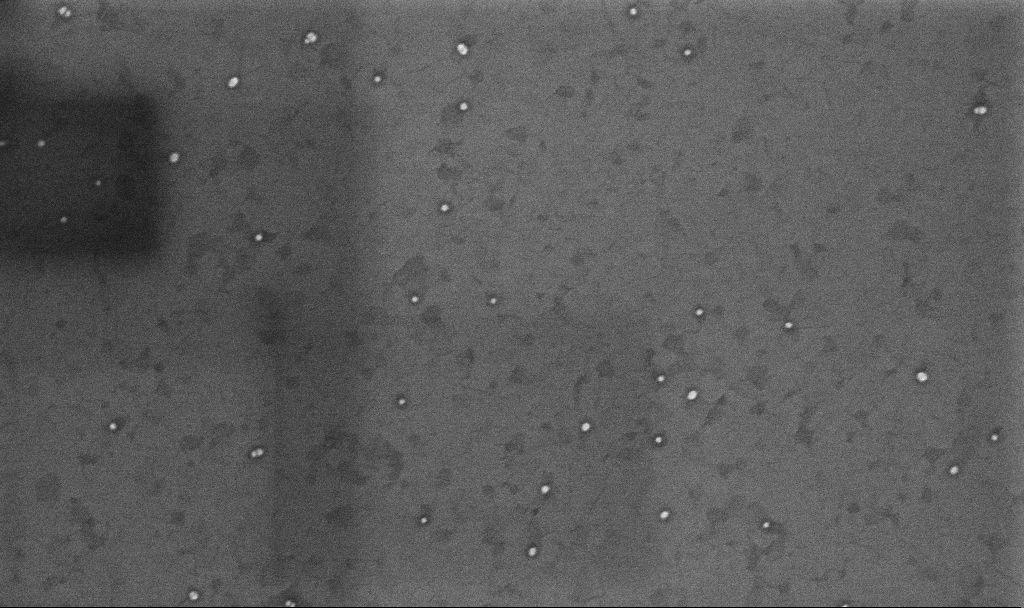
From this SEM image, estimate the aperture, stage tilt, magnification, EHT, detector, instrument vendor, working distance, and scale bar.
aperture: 30 µm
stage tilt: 0°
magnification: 100.38 K X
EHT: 10 kV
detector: InLens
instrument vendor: Zeiss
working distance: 3.3 mm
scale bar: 200 nm